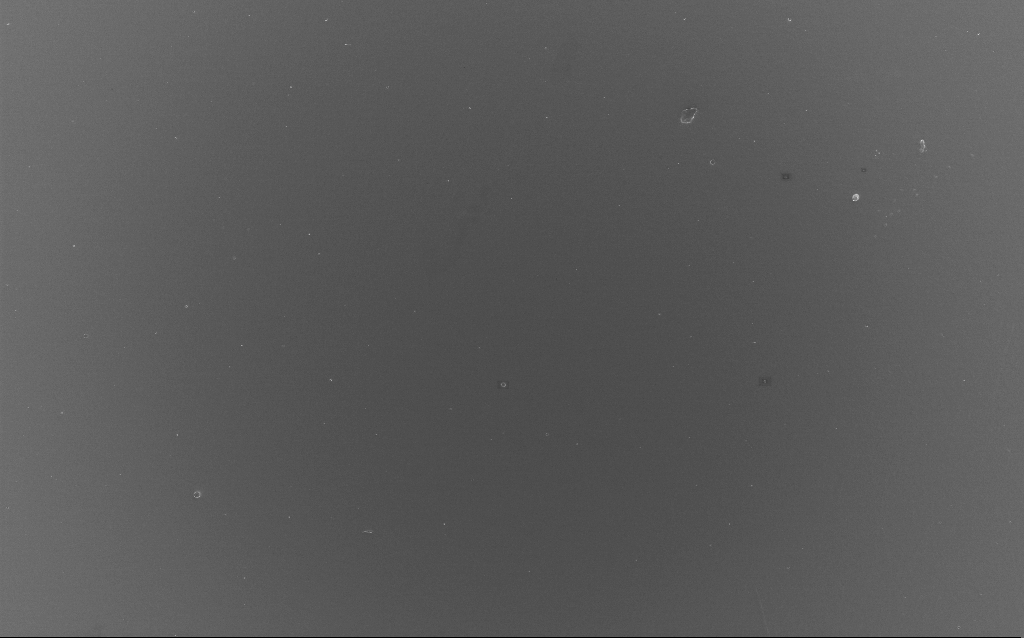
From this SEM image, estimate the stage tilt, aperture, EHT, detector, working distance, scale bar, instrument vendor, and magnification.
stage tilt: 0°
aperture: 30 µm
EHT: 10 kV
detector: InLens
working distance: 2 mm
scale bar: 100000 nm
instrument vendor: Zeiss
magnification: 0.691 K X